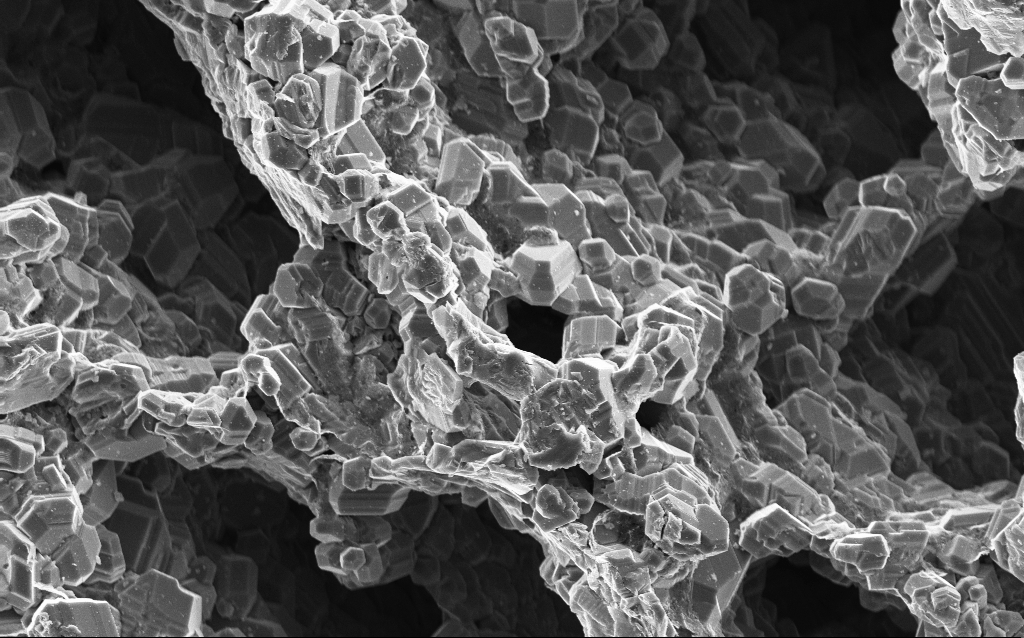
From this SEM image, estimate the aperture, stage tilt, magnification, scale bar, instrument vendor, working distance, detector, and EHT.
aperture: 30 µm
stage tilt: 0°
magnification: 3 K X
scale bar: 10000 nm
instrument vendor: Zeiss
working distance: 3 mm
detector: InLens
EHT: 10 kV